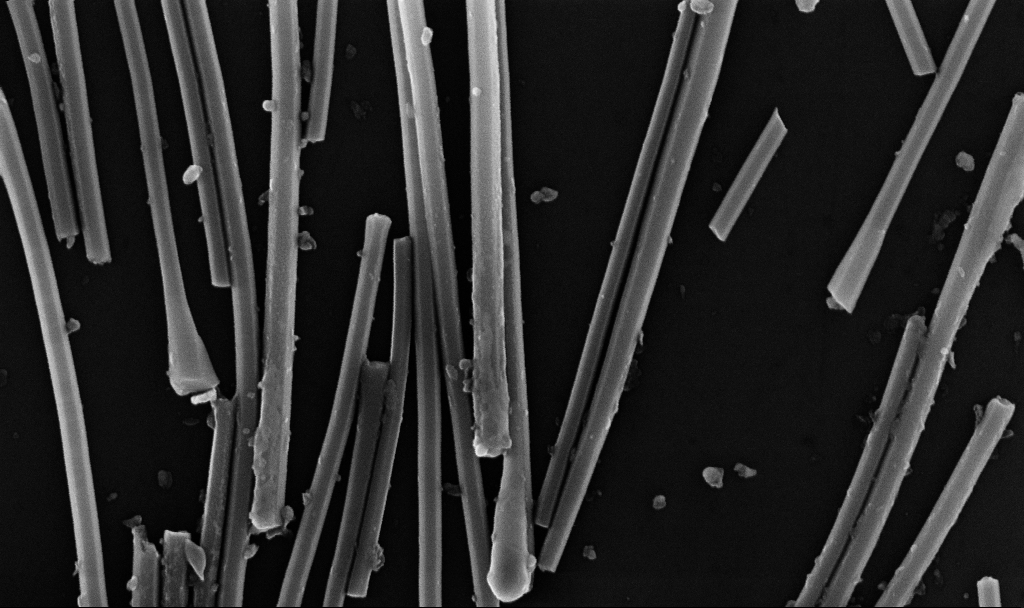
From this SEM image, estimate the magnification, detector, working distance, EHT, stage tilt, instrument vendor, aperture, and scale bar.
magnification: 83.22 K X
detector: InLens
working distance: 6.7 mm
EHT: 10 kV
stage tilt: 0°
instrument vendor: Zeiss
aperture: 30 µm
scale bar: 200 nm